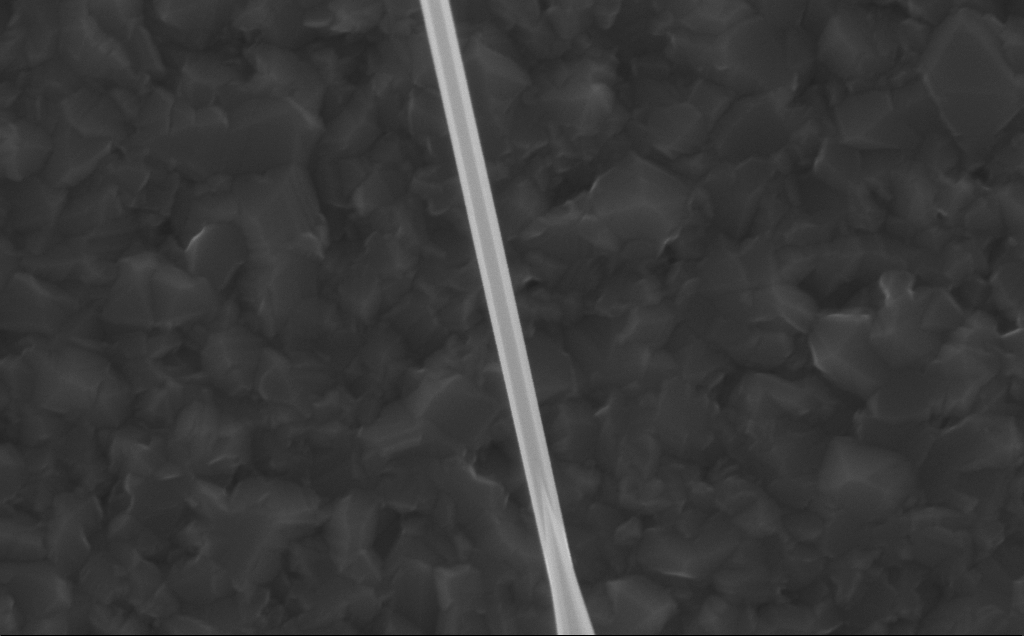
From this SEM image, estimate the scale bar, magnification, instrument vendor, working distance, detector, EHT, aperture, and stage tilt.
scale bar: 200 nm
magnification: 78.35 K X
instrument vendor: Zeiss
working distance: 5 mm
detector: InLens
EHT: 10 kV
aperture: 30 µm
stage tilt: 0°